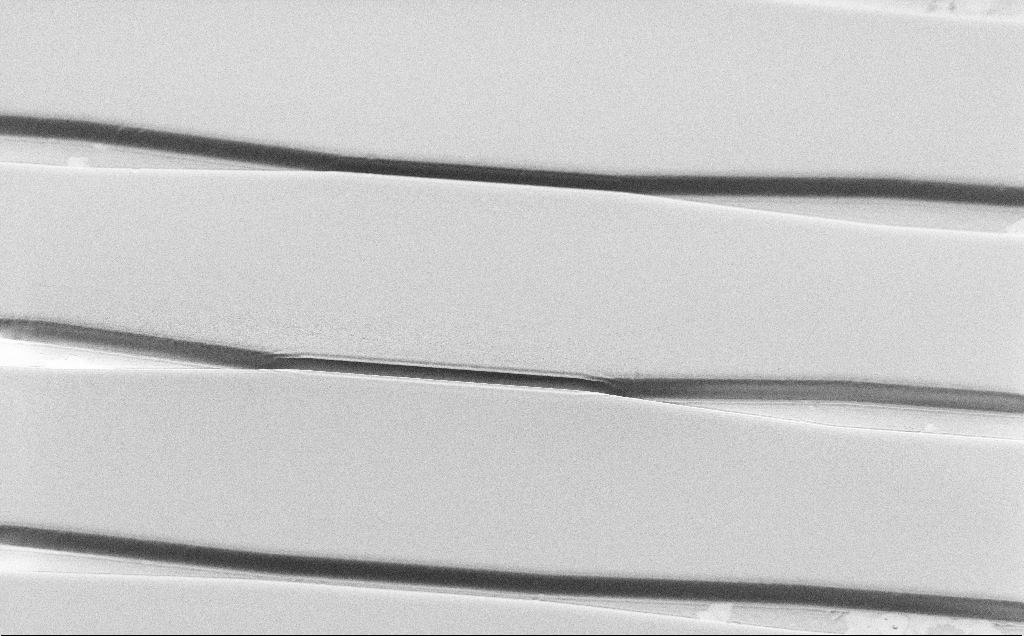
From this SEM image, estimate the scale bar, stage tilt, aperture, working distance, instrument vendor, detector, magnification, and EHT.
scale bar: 20000 nm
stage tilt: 45°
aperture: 30 µm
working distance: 7 mm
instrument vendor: Zeiss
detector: SE2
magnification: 1.01 K X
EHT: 1 kV